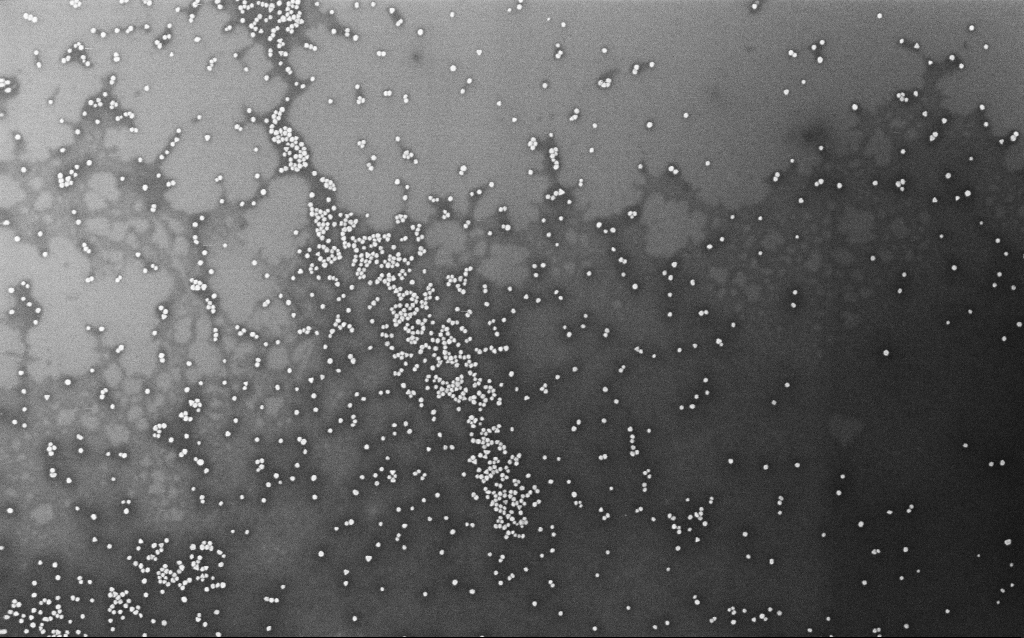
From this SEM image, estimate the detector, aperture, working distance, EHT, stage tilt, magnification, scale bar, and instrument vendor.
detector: InLens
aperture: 30 µm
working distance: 1.9 mm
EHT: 10 kV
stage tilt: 0°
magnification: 100 K X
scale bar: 200 nm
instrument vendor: Zeiss